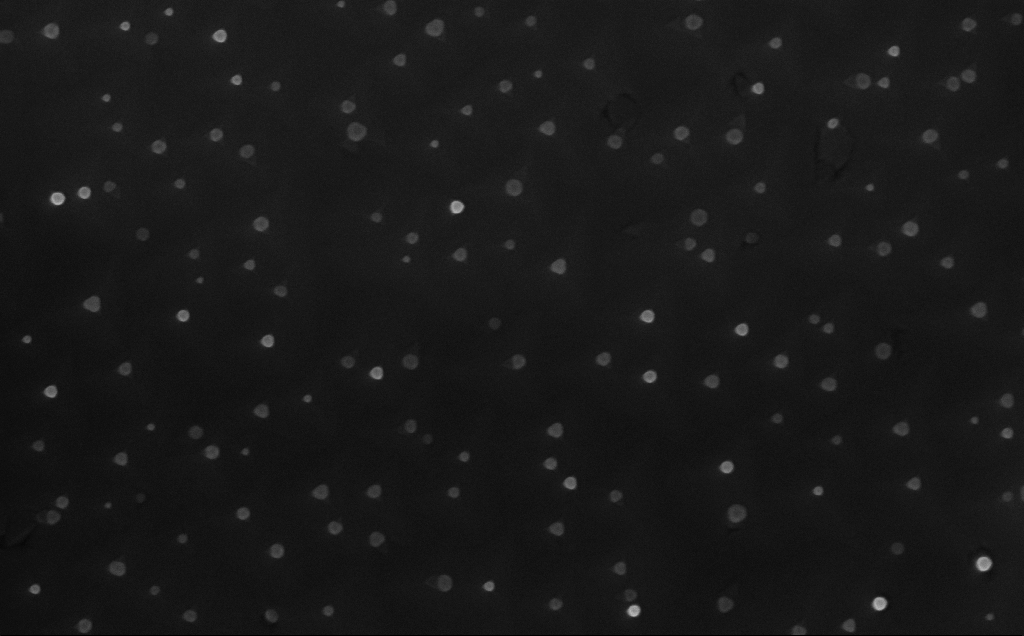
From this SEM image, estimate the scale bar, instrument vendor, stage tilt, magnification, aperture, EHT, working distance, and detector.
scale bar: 200 nm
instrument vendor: Zeiss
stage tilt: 0°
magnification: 80 K X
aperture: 30 µm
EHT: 10 kV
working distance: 4 mm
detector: InLens